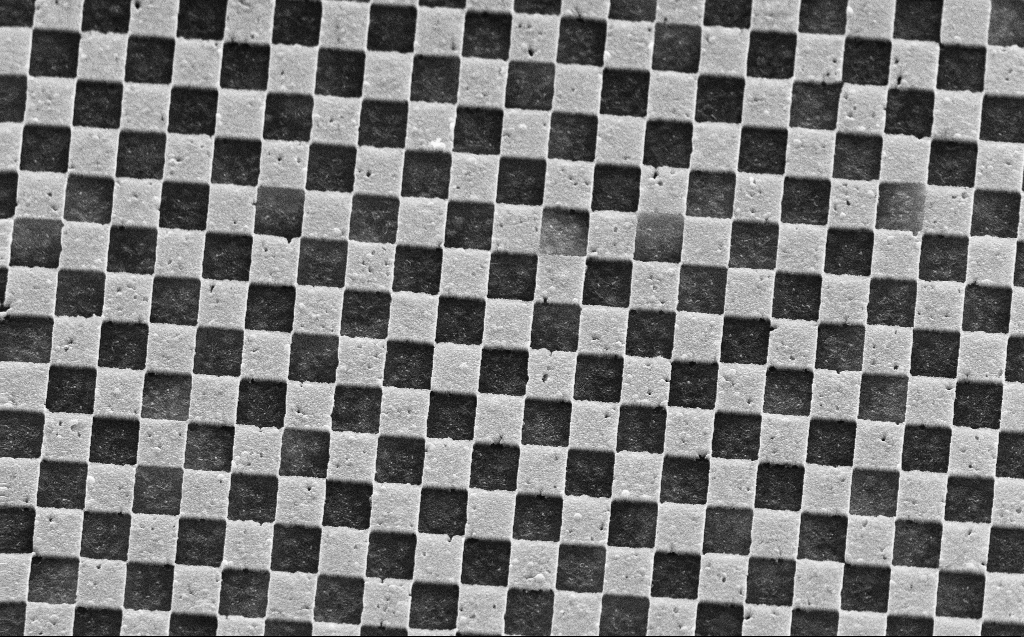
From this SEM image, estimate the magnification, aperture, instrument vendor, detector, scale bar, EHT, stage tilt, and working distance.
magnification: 17.86 K X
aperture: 30 µm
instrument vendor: Zeiss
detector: SE2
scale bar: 1000 nm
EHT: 30 kV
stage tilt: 45°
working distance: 5 mm